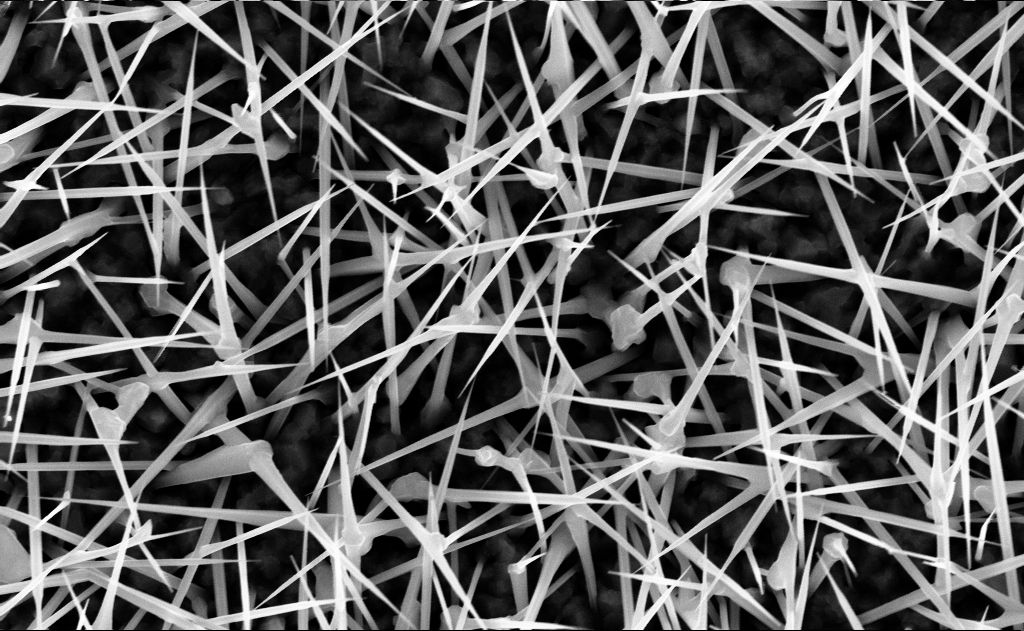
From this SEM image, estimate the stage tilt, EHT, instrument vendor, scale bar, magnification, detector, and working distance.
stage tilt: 0°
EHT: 10 kV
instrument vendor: Zeiss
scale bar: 1000 nm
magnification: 40 K X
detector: InLens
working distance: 9 mm